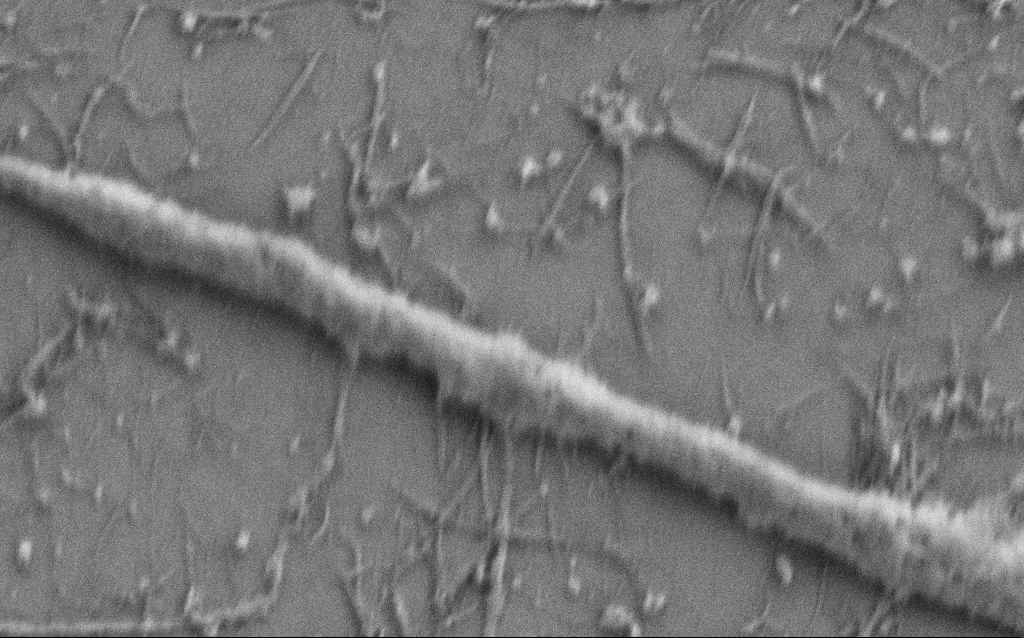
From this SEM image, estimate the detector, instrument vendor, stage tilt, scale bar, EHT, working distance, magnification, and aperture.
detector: SE2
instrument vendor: Zeiss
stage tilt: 0°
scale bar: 1000 nm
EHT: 0.8 kV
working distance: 5.8 mm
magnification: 50 K X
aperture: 30 µm